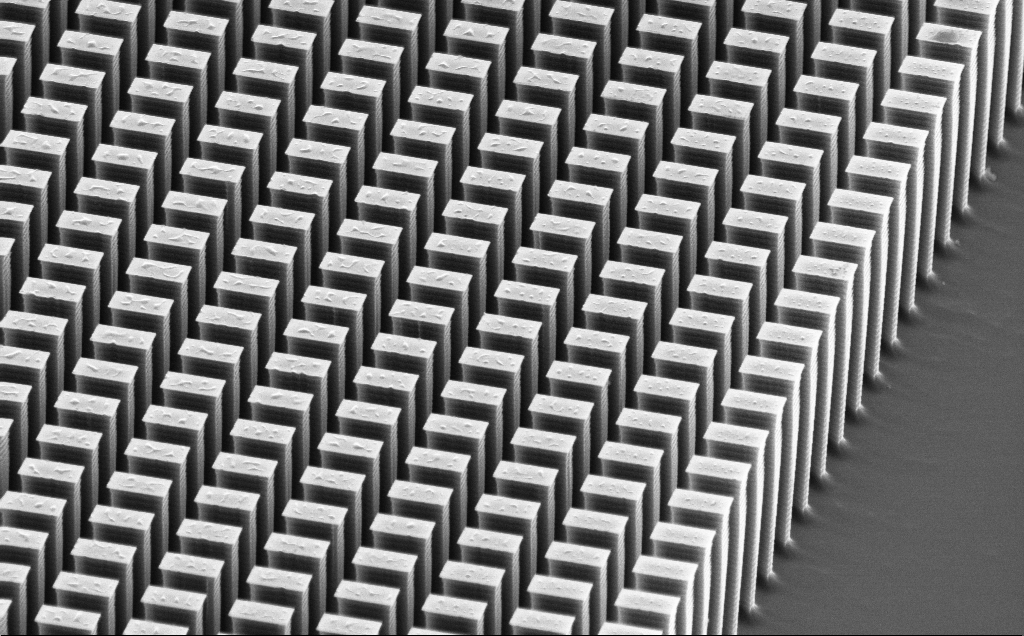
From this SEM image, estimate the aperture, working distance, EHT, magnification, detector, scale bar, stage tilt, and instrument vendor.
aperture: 30 µm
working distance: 15 mm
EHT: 10 kV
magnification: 5.61 K X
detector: SE2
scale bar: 10000 nm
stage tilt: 60.2°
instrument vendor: Zeiss